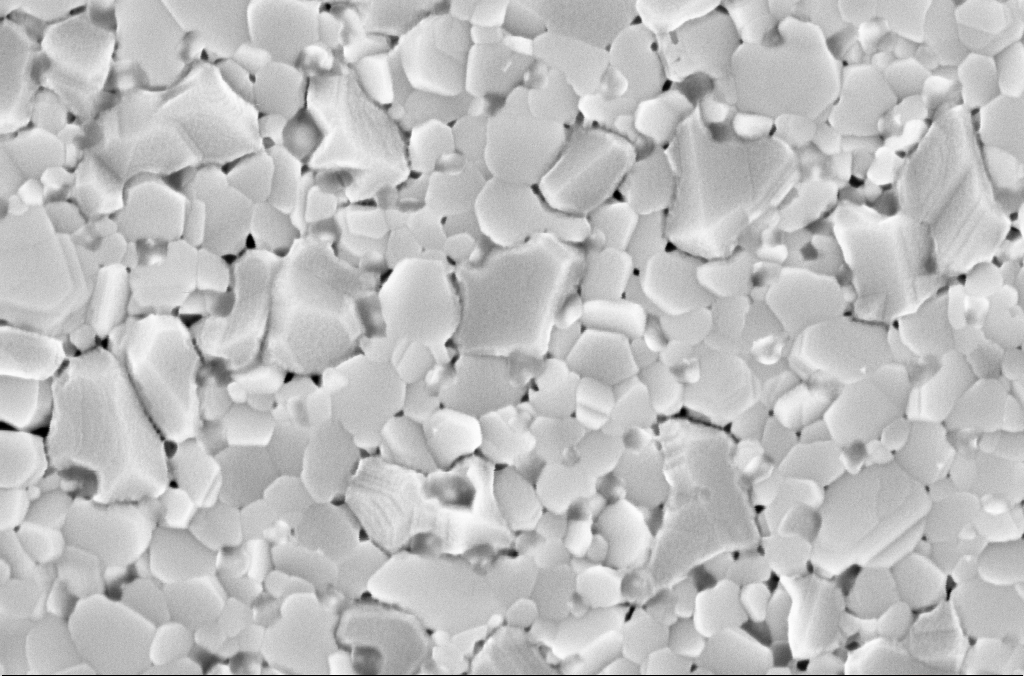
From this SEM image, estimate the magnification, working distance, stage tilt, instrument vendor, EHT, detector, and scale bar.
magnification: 100 K X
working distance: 3 mm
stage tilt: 0°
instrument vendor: Zeiss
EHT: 5 kV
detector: InLens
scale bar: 200 nm